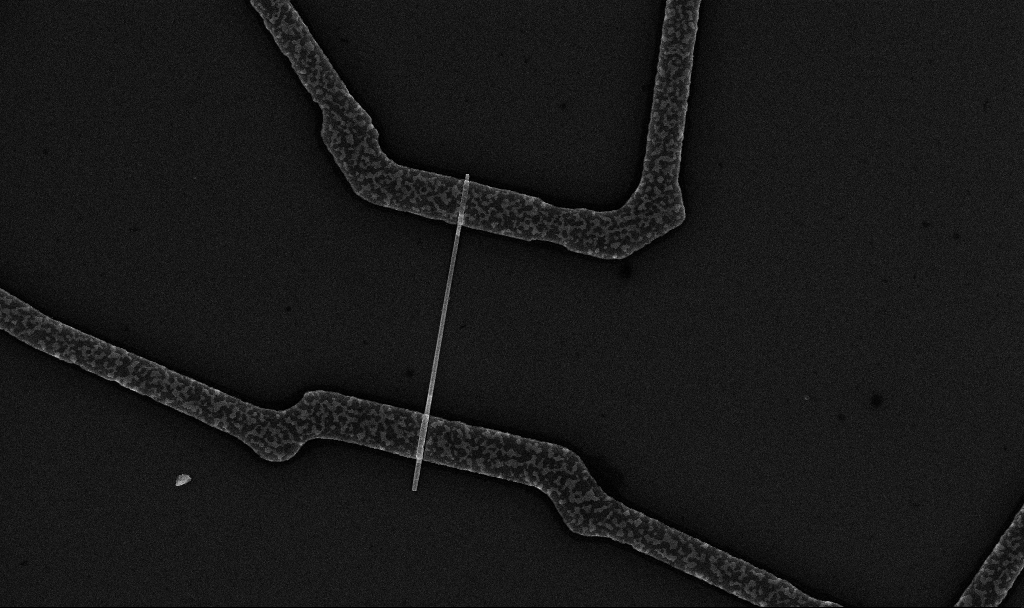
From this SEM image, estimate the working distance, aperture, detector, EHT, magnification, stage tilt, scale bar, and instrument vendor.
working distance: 6.7 mm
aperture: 30 µm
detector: InLens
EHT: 10 kV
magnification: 18.2 K X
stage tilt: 0°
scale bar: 1000 nm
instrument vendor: Zeiss